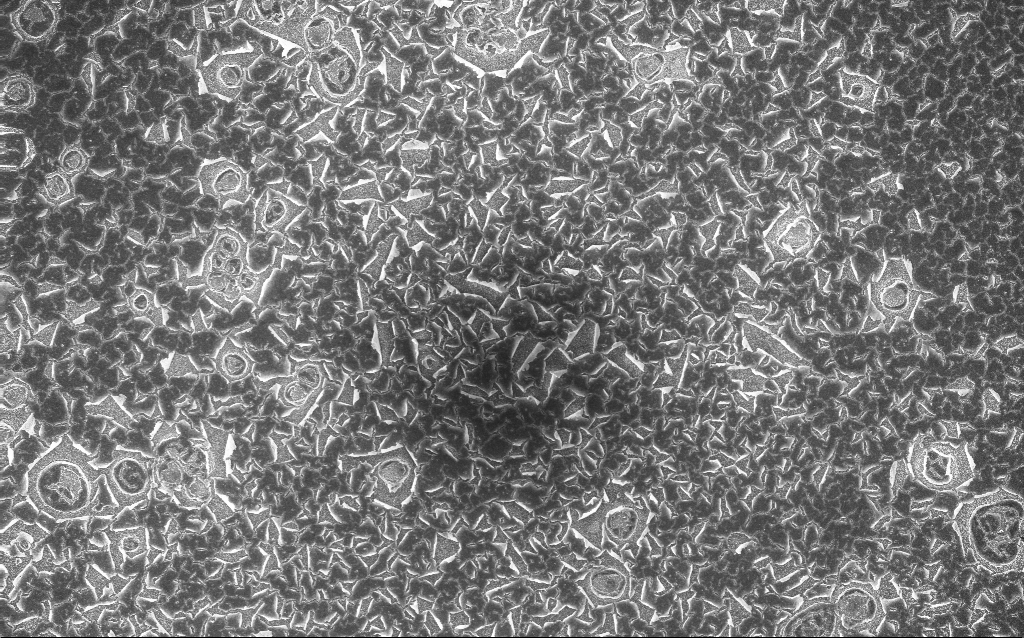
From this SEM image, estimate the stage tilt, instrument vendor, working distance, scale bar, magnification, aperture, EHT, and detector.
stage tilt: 0°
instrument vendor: Zeiss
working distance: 2.8 mm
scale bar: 100000 nm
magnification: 0.2 K X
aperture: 30 µm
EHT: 10 kV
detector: InLens